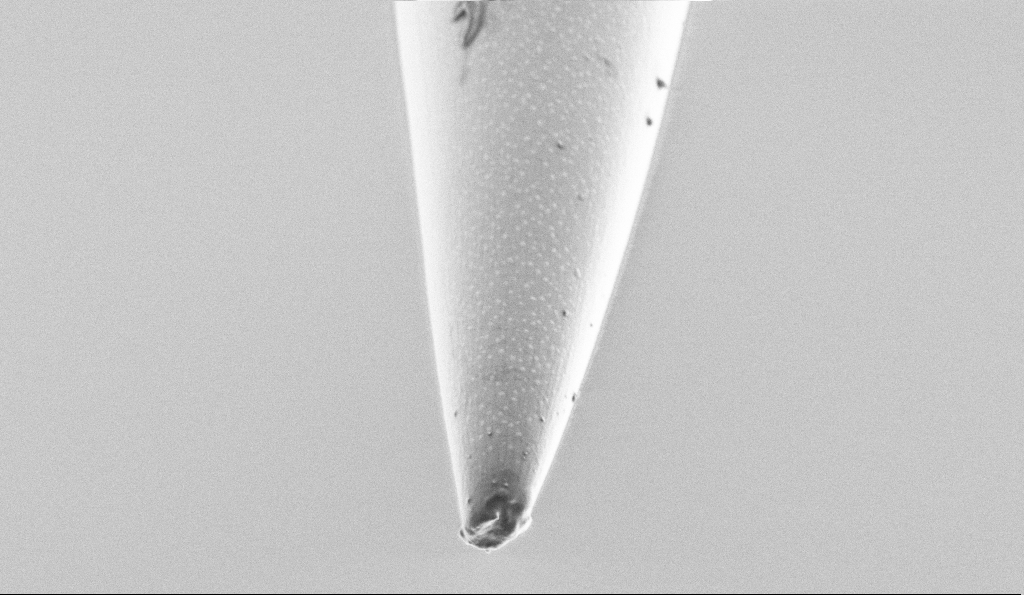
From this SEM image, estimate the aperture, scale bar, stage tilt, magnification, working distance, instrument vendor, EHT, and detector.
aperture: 30 µm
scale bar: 2000 nm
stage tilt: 45°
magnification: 15 K X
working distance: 7.5 mm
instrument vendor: Zeiss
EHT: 1 kV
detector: SE2